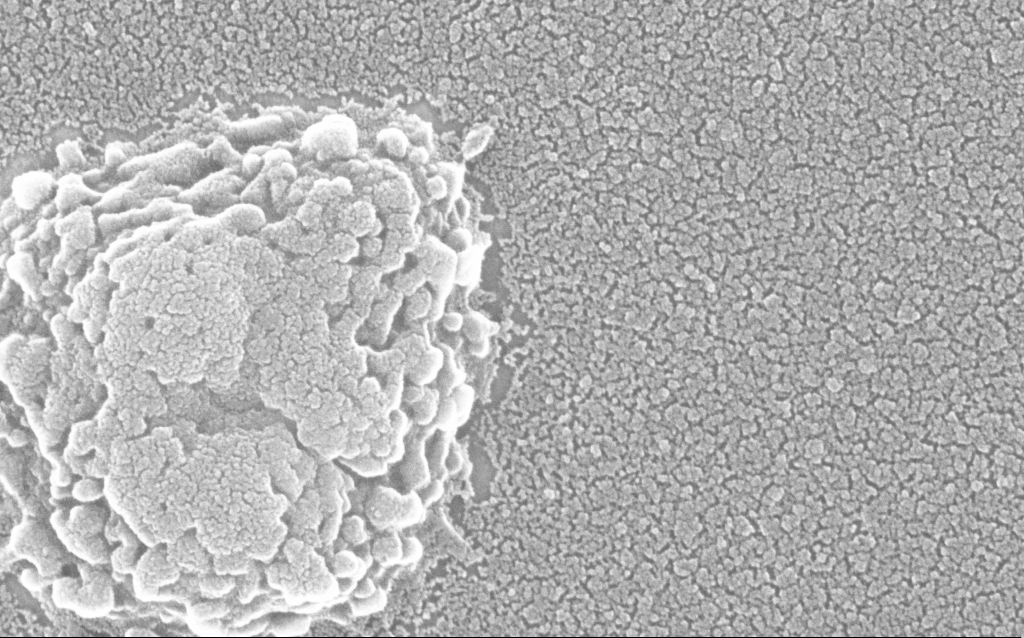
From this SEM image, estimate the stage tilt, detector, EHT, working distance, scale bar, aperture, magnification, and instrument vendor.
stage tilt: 0°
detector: InLens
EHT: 20 kV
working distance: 1.8 mm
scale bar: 100 nm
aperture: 30 µm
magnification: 300 K X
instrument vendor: Zeiss